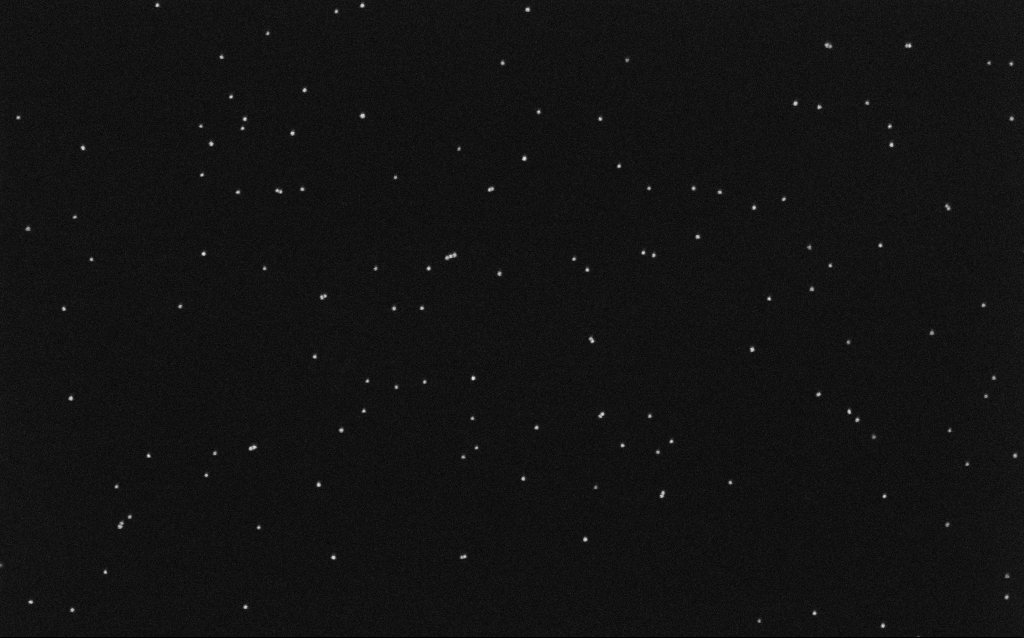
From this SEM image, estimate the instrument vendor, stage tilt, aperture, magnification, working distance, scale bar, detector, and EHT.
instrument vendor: Zeiss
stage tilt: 0°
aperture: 30 µm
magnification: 100 K X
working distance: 6.5 mm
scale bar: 200 nm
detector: InLens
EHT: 10 kV